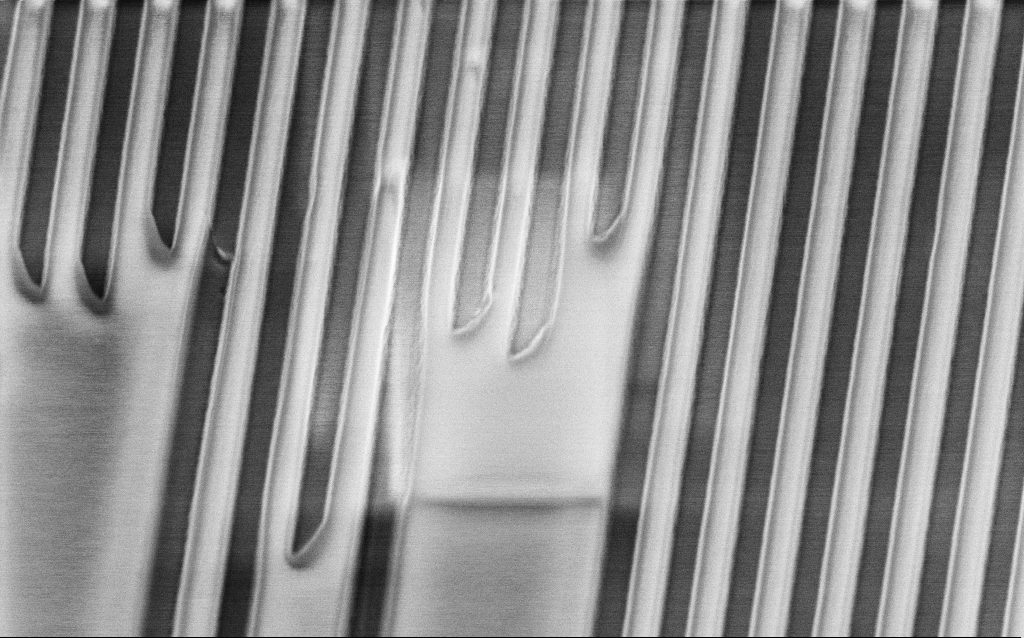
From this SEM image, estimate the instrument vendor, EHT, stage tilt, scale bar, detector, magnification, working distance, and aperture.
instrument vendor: Zeiss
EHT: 2 kV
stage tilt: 45°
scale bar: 1000 nm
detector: InLens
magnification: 46.4 K X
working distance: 4.1 mm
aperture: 30 µm